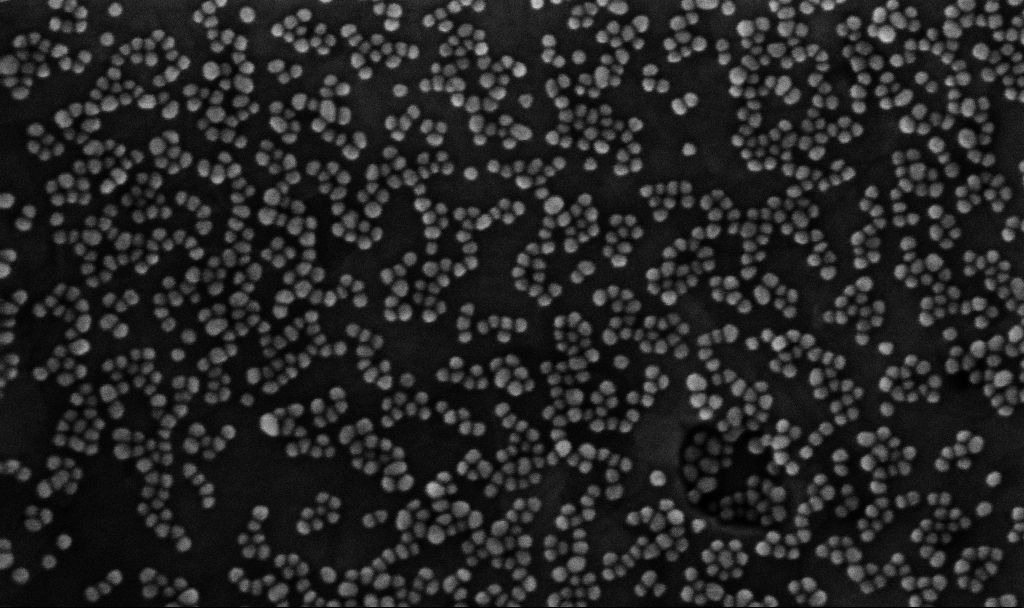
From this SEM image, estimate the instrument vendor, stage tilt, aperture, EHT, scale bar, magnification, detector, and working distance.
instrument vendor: Zeiss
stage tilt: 0°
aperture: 30 µm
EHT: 10 kV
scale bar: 200 nm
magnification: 300 K X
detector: InLens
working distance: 3.3 mm